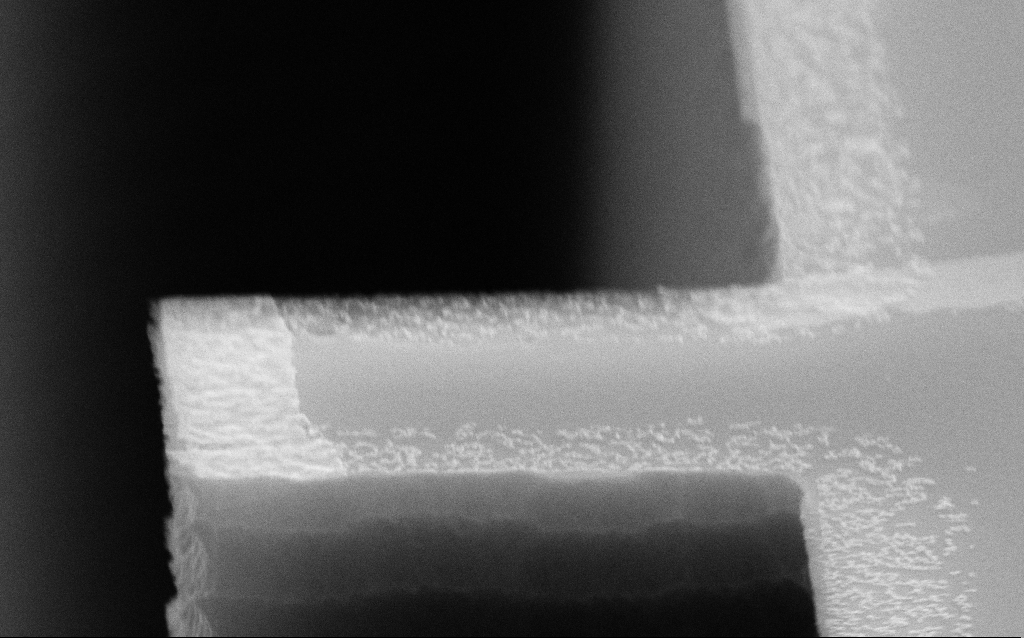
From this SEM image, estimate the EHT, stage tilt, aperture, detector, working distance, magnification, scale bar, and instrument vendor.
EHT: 10 kV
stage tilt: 70°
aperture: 30 µm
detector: SE2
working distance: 7.4 mm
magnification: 96.81 K X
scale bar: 200 nm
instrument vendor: Zeiss